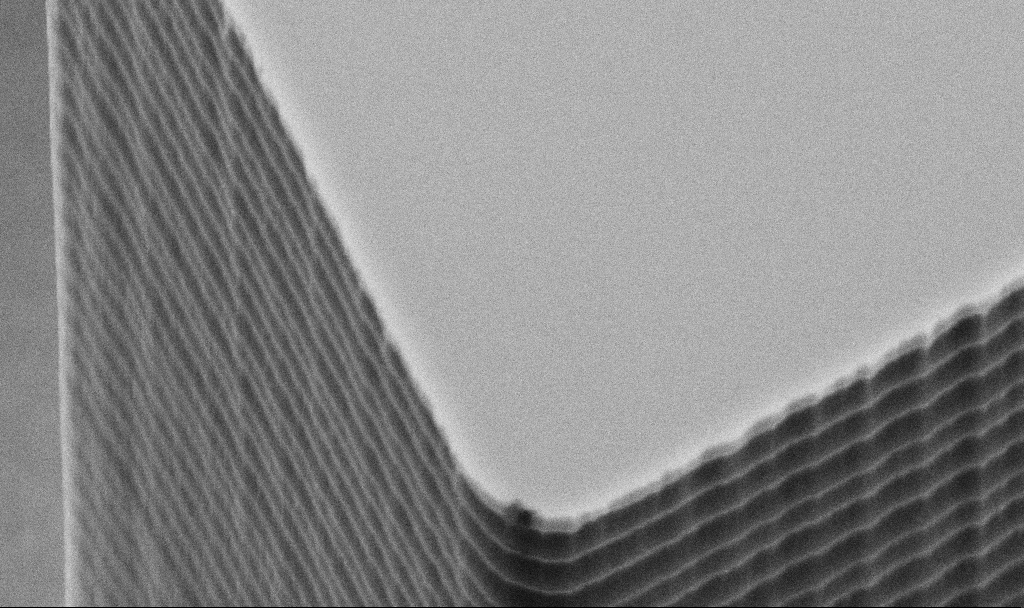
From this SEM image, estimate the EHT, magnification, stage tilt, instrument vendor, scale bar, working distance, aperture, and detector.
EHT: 5 kV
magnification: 41.09 K X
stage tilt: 45°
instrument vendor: Zeiss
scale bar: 1000 nm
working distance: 6.3 mm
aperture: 30 µm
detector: SE2